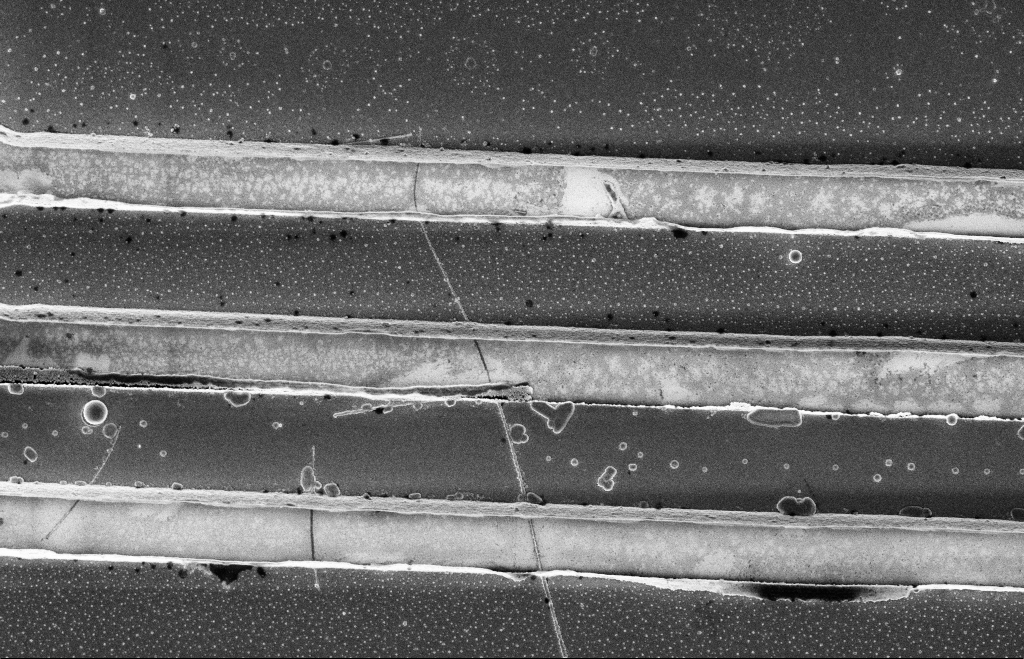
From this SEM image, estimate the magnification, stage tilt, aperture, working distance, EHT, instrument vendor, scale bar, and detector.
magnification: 10.72 K X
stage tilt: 0°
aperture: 20 µm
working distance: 12 mm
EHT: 5 kV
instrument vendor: Zeiss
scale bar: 2000 nm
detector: InLens